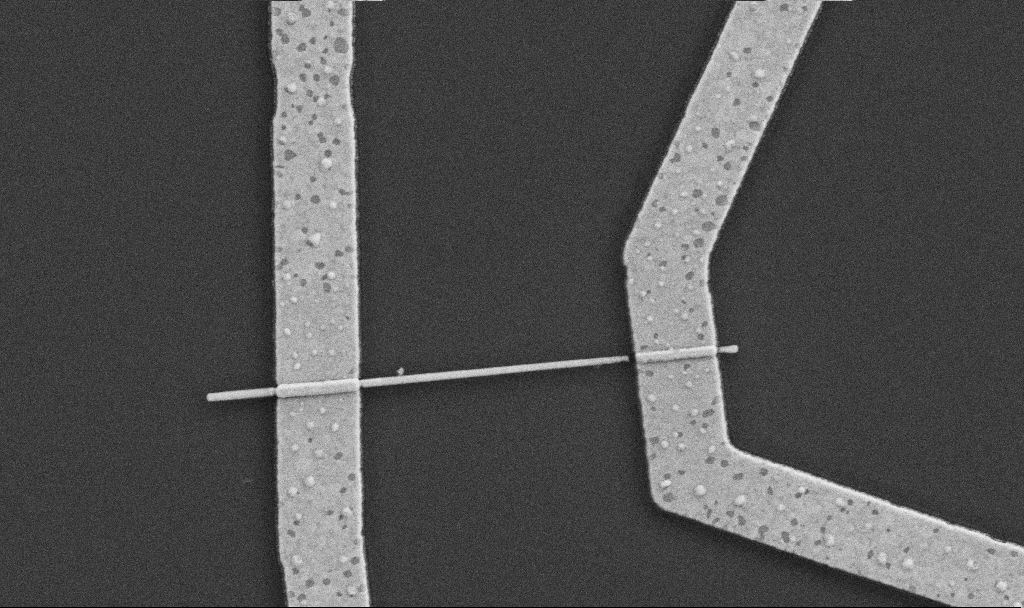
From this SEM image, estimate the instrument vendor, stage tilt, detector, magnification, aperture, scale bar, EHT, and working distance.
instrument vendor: Zeiss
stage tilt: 0°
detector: SE2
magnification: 30 K X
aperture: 30 µm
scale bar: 1000 nm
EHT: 5 kV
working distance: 8.7 mm